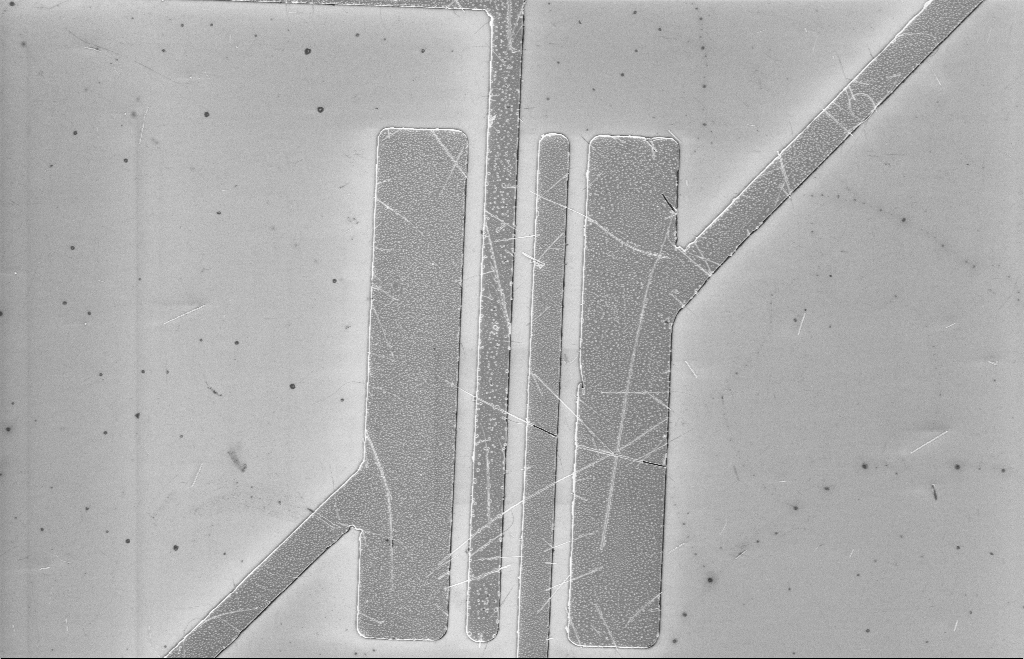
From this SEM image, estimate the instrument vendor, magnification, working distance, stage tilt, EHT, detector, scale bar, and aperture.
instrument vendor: Zeiss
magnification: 3.13 K X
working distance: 8 mm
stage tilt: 0°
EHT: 5 kV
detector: InLens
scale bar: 10000 nm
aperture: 20 µm